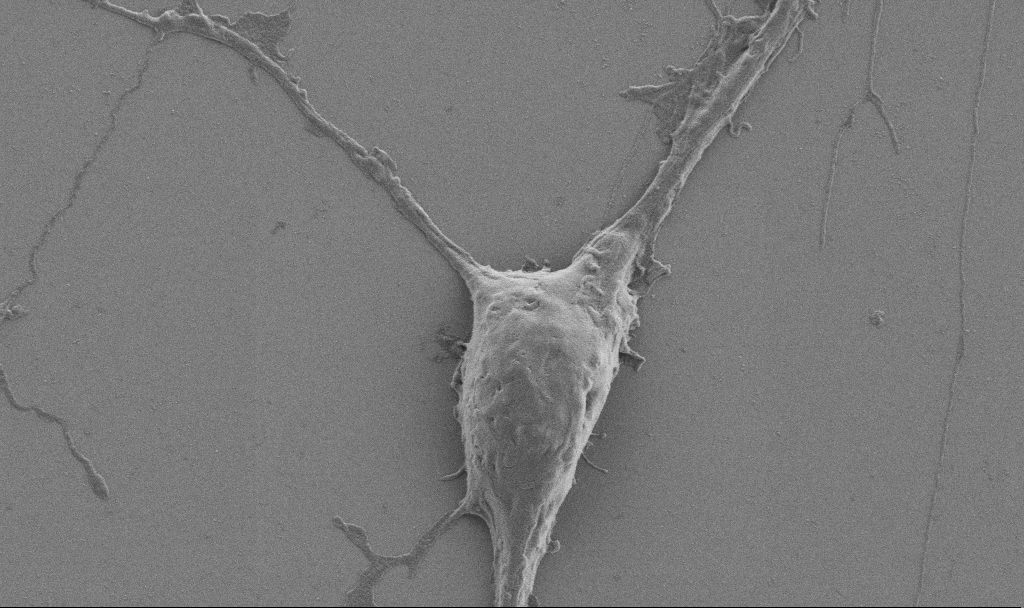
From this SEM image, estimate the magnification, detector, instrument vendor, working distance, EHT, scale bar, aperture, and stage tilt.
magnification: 10 K X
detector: SE2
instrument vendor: Zeiss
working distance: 6.9 mm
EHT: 0.9 kV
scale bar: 2000 nm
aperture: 30 µm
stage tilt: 0°